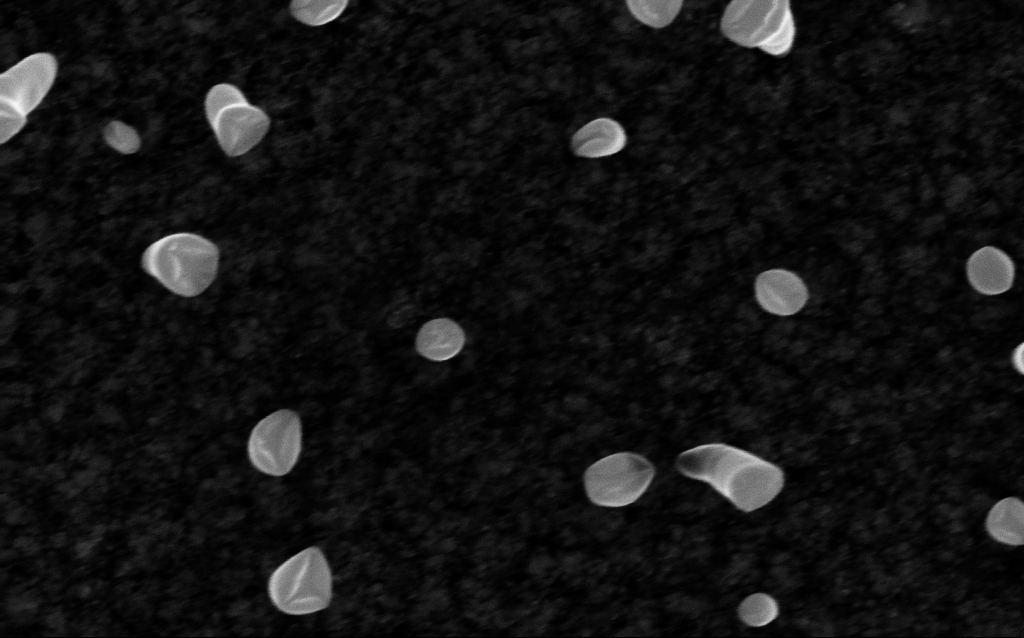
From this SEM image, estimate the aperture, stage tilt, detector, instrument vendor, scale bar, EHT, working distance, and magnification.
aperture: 30 µm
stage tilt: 0°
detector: InLens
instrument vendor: Zeiss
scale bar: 100 nm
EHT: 5 kV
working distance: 2 mm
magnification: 200 K X